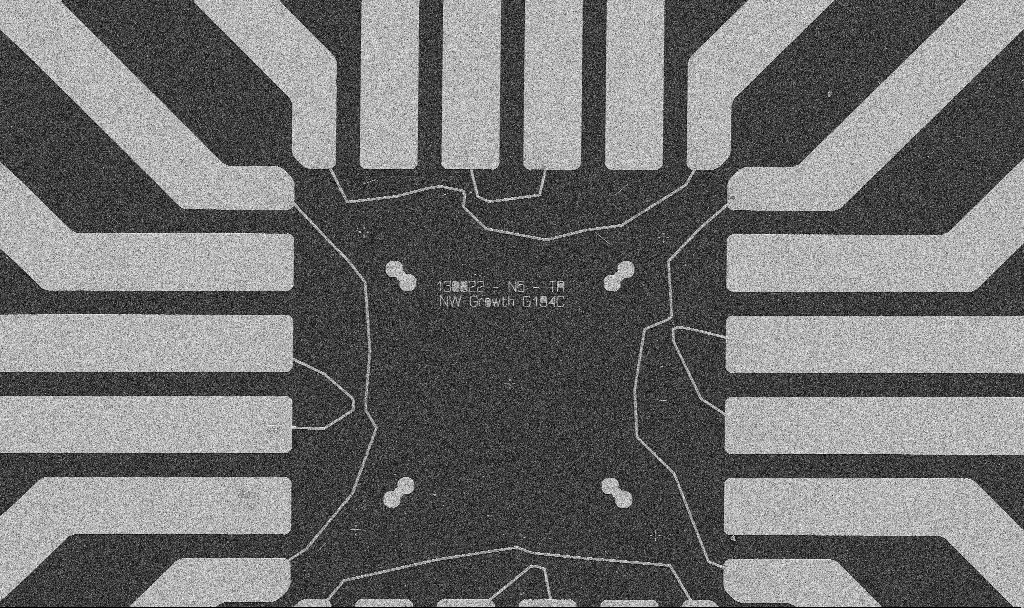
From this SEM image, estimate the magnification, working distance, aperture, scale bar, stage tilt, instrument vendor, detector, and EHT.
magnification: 1 K X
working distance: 8.7 mm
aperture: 30 µm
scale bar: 20000 nm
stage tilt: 0°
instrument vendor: Zeiss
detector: SE2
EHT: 5 kV